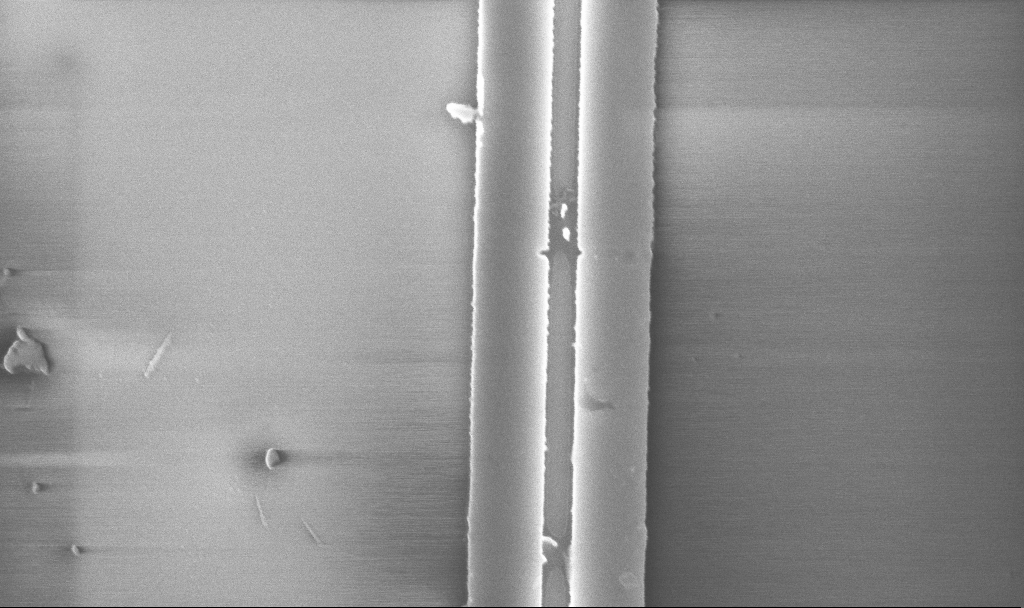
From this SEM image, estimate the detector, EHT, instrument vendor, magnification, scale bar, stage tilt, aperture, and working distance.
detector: InLens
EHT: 5 kV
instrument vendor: Zeiss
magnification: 52.86 K X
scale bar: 1000 nm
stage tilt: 0°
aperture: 30 µm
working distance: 10 mm